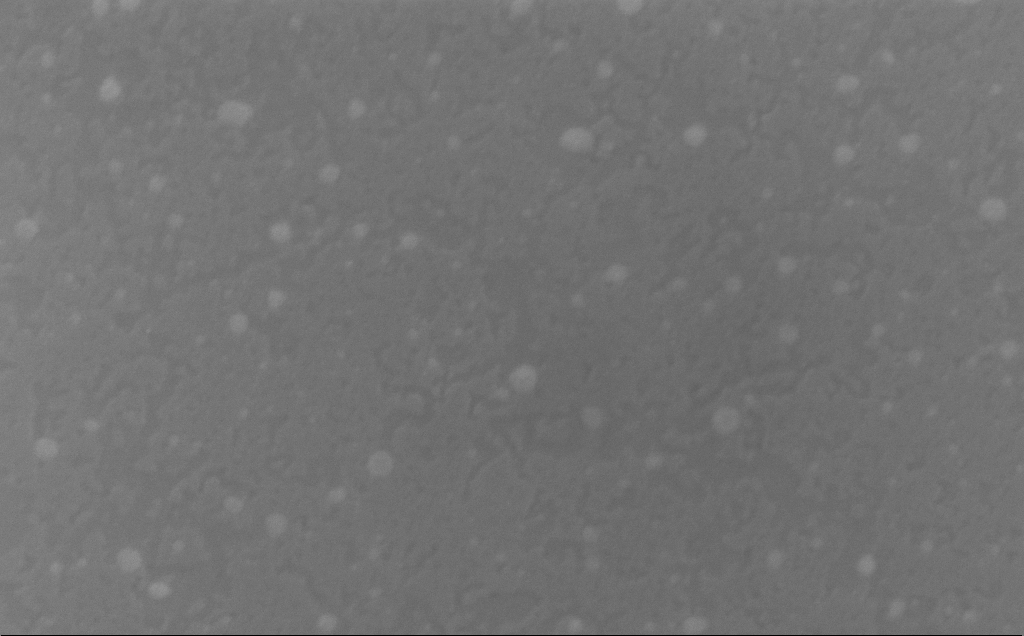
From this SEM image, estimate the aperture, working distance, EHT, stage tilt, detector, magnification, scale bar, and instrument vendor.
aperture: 30 µm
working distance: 5 mm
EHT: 10 kV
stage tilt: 15.5°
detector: InLens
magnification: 259.92 K X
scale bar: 100 nm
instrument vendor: Zeiss